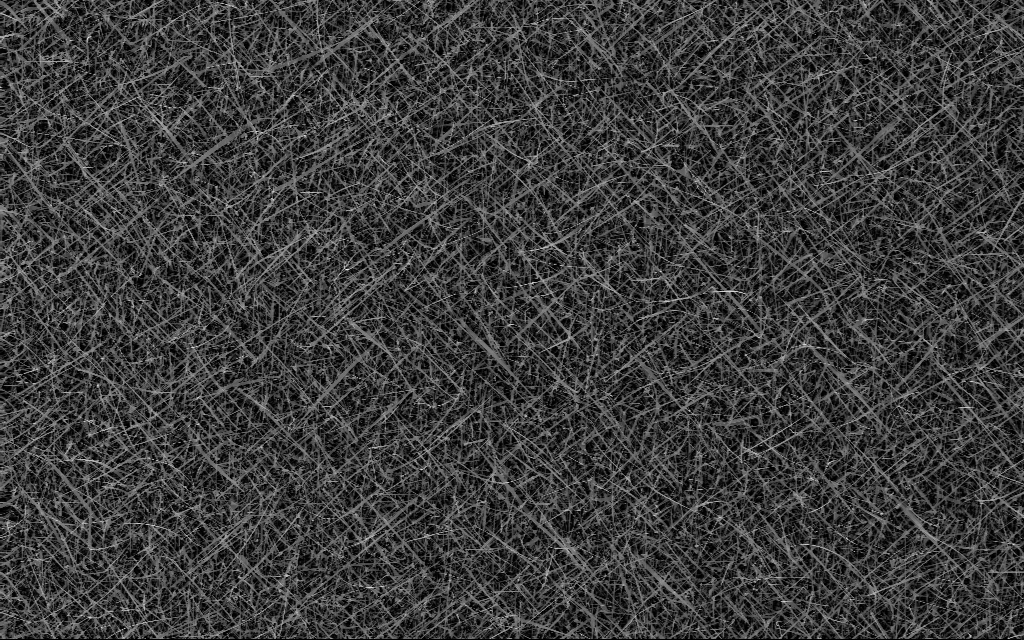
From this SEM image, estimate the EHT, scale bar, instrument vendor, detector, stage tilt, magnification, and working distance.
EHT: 10 kV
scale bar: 10000 nm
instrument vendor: Zeiss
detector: InLens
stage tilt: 0°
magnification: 5 K X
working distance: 7 mm